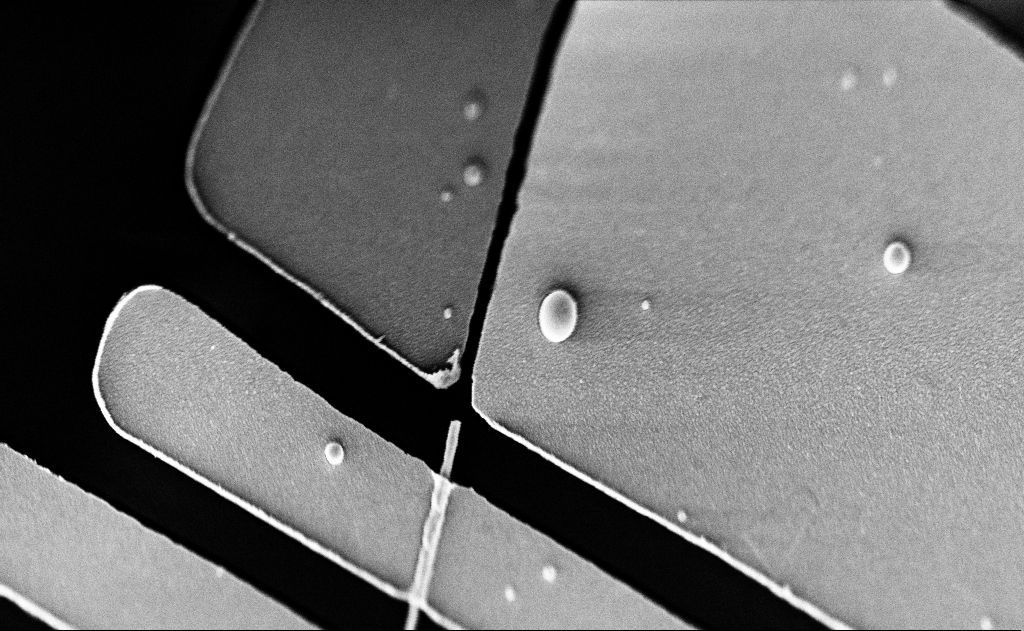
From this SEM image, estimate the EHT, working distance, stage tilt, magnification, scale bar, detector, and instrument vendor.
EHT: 5 kV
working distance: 20 mm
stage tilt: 0°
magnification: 16.18 K X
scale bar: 2000 nm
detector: SE2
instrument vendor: Zeiss